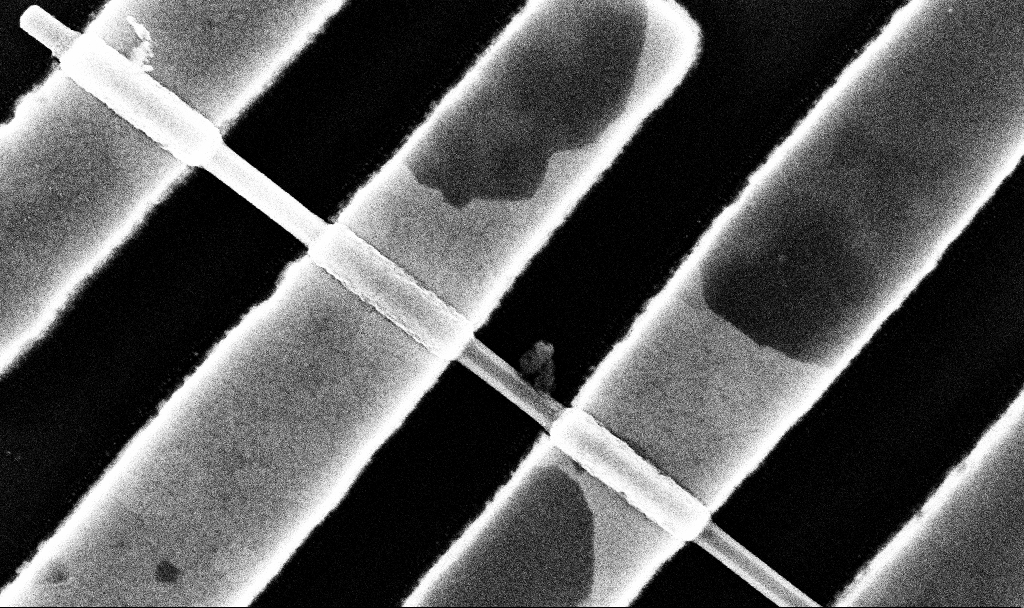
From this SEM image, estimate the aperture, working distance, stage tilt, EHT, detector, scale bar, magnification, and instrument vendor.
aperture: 30 µm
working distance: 6.8 mm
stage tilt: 0°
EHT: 10 kV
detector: InLens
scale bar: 200 nm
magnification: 106.01 K X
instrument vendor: Zeiss